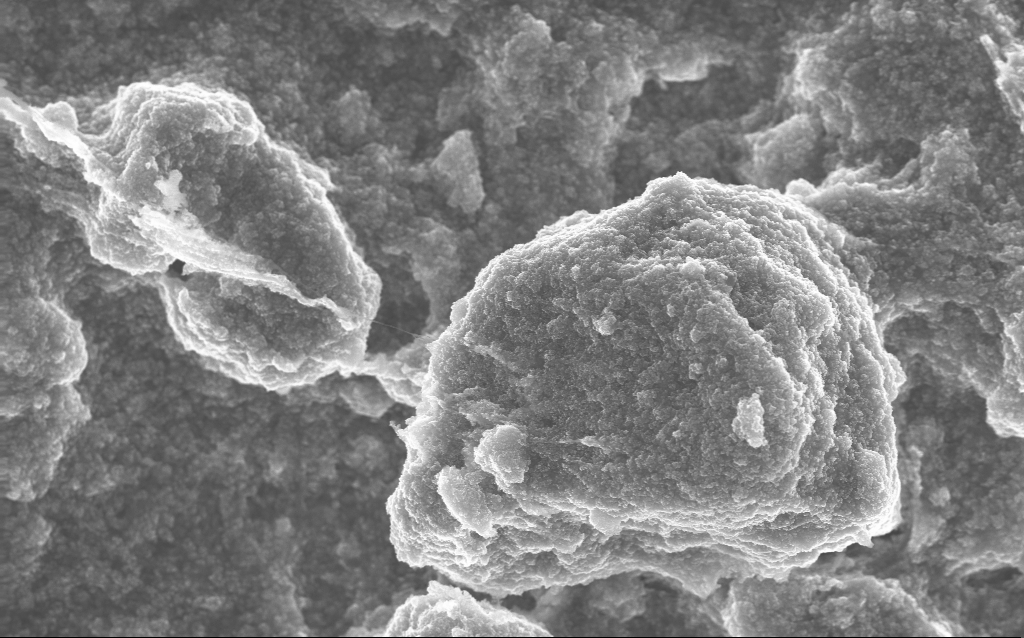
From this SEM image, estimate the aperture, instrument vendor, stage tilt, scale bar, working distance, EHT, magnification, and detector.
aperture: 30 µm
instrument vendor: Zeiss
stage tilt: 0°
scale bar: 1000 nm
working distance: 2.7 mm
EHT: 10 kV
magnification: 15.33 K X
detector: InLens